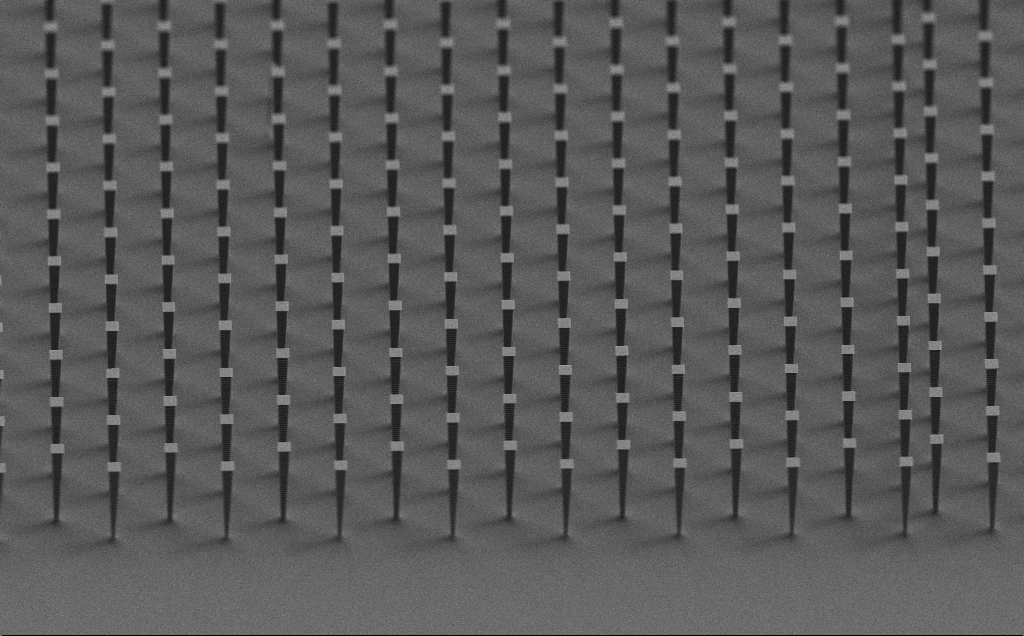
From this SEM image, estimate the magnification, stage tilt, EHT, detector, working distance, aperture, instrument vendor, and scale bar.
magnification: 2.29 K X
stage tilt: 60°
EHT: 3 kV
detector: SE2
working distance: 7 mm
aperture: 30 µm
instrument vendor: Zeiss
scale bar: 20000 nm